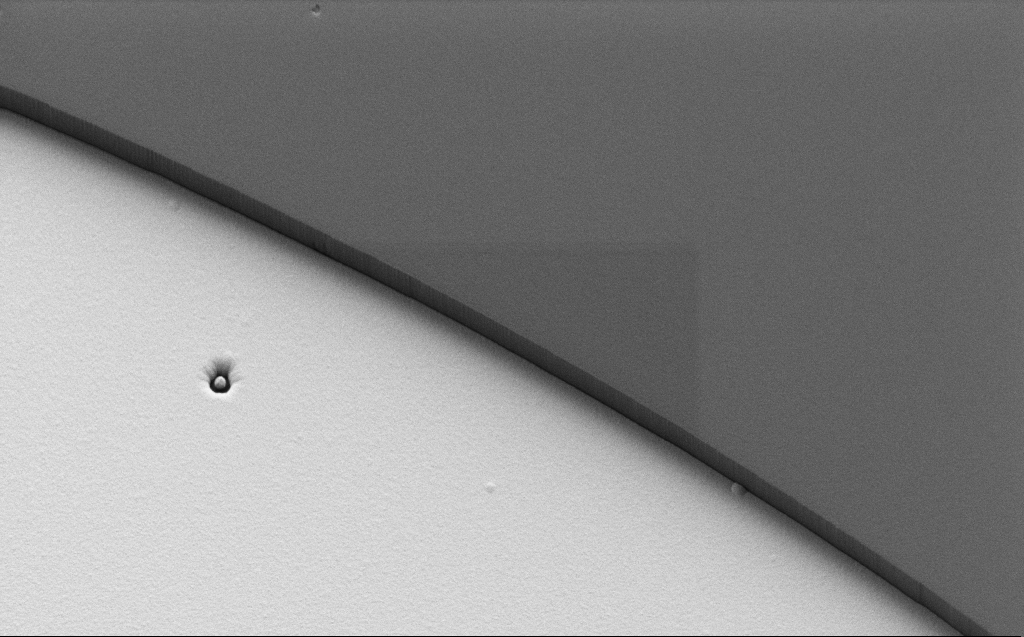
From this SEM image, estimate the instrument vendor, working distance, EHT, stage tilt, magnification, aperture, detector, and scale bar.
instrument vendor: Zeiss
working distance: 6 mm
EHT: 1.1 kV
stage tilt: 30°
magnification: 5.93 K X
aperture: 30 µm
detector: SE2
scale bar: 10000 nm